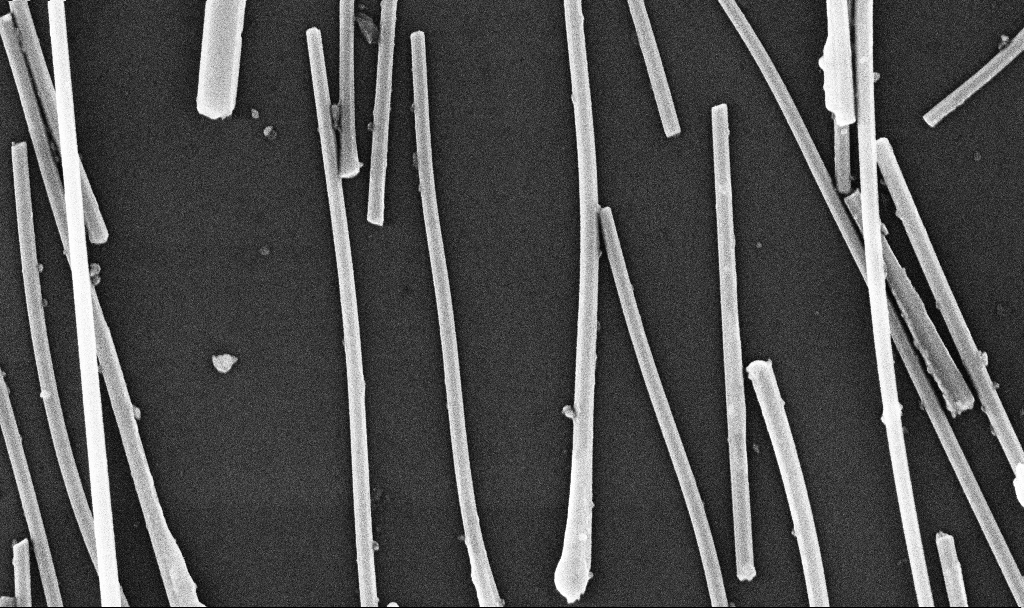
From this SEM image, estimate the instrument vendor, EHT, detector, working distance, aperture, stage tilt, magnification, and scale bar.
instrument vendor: Zeiss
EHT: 10 kV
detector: InLens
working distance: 6.7 mm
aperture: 30 µm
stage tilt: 0°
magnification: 55.86 K X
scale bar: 1000 nm